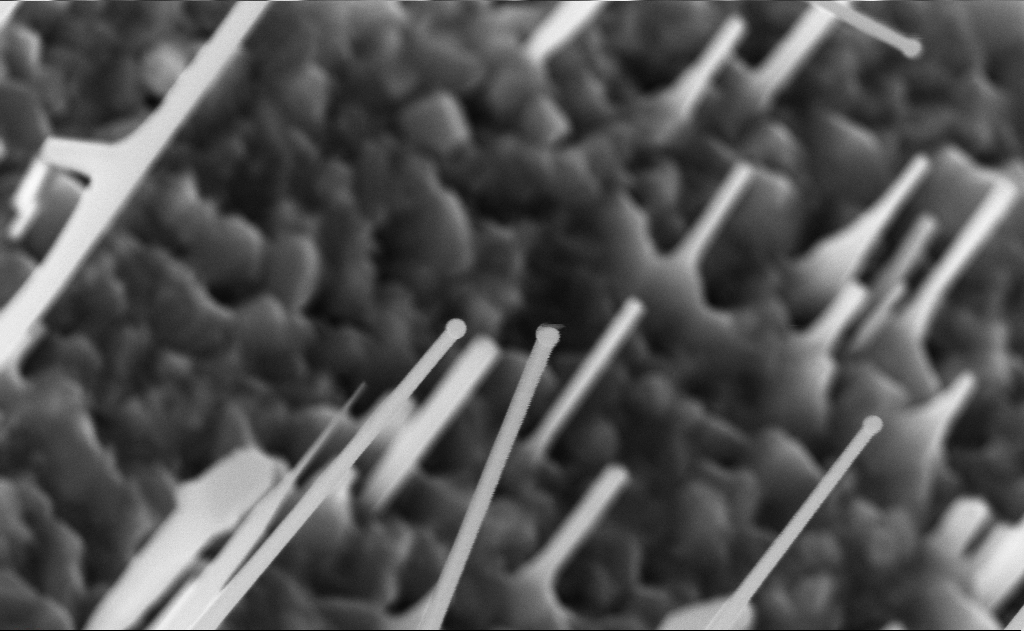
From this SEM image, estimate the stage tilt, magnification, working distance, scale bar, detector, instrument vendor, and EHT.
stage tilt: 0°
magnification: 80 K X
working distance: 10 mm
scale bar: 200 nm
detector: InLens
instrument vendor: Zeiss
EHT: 10 kV